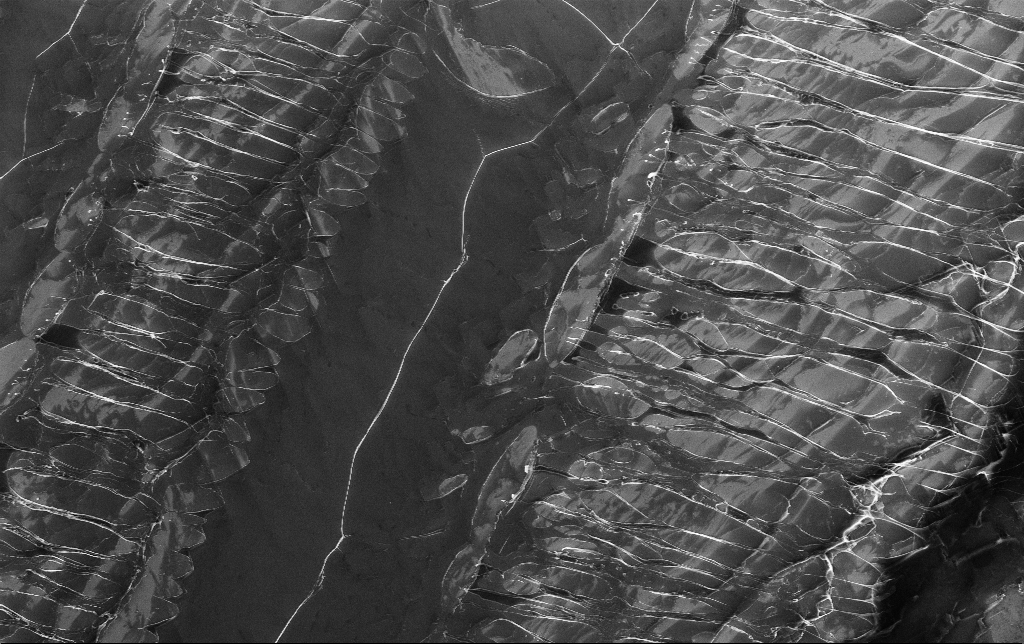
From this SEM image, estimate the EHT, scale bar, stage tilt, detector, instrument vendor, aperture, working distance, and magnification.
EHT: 5 kV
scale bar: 10000 nm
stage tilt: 0°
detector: InLens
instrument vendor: Zeiss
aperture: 30 µm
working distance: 3.8 mm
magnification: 1.75 K X